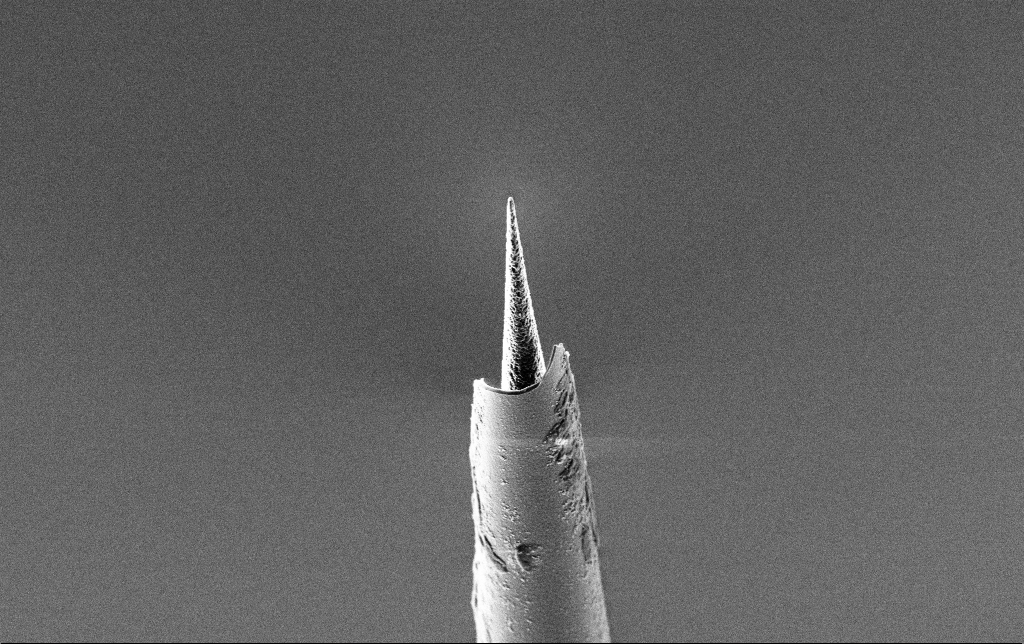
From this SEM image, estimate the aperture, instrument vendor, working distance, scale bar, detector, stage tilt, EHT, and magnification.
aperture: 30 µm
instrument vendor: Zeiss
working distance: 7.4 mm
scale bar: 2000 nm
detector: SE2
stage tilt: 45°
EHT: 2 kV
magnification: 10 K X